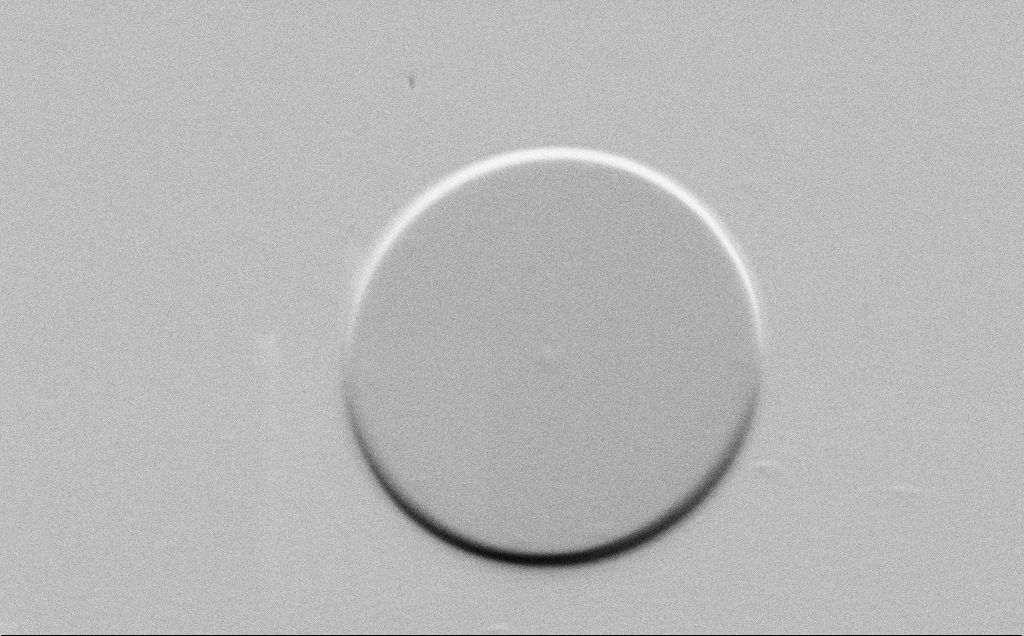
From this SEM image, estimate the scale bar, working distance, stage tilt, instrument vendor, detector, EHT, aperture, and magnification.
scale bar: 20000 nm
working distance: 4 mm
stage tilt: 45°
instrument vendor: Zeiss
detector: SE2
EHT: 1.5 kV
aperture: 30 µm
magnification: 2.6 K X